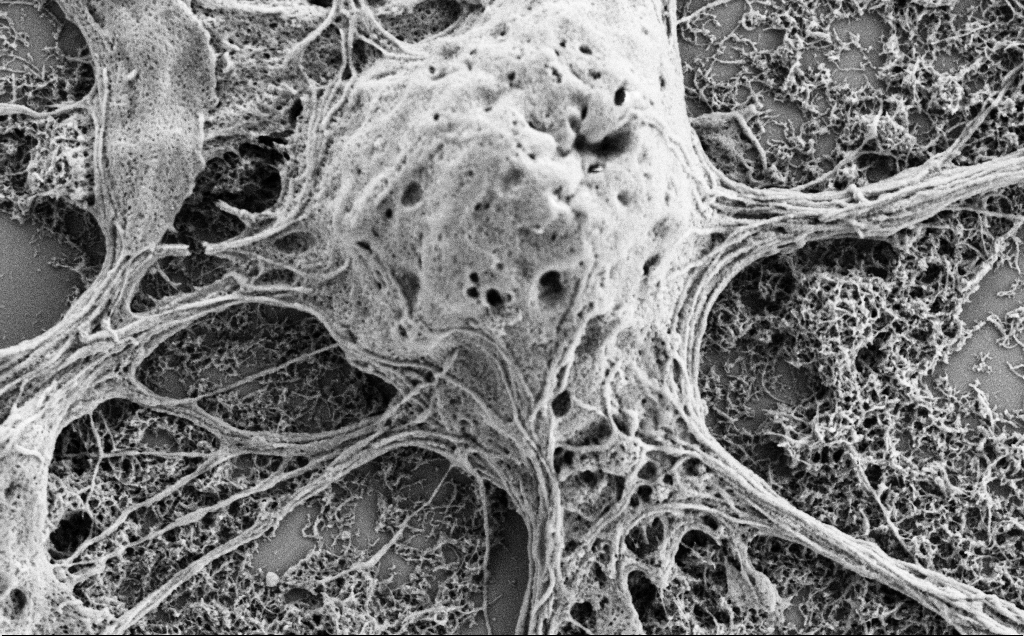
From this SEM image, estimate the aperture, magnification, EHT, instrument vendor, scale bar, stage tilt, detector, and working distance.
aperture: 30 µm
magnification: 20 K X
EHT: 2 kV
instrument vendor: Zeiss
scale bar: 2000 nm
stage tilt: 0°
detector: SE2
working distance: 7 mm